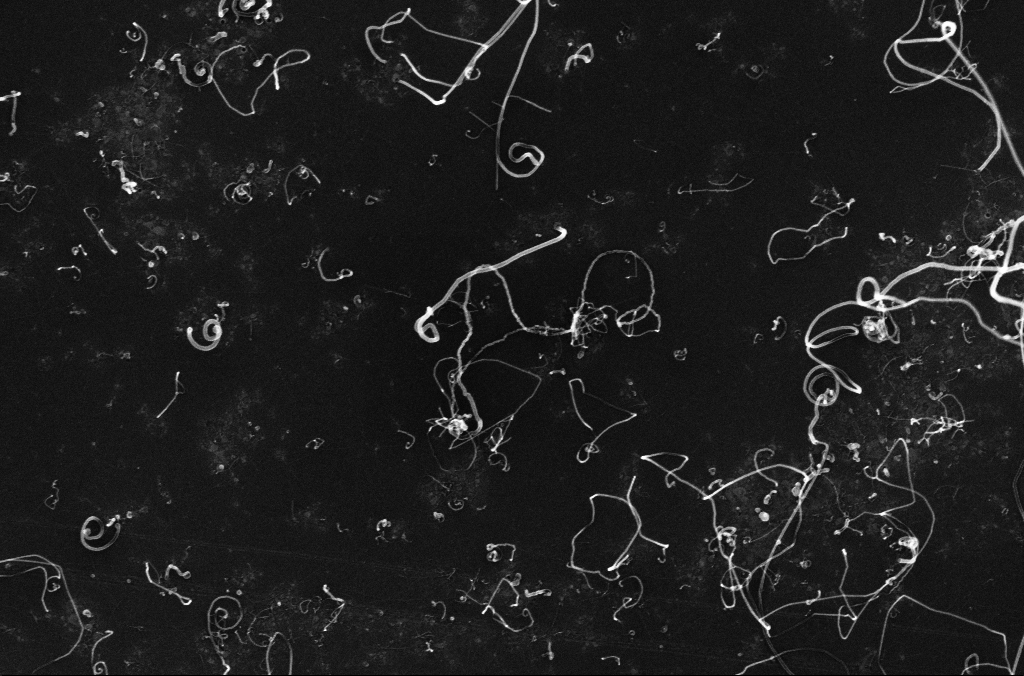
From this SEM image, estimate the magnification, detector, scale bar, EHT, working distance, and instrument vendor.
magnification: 60 K X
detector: InLens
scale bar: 1000 nm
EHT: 10 kV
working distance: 3.3 mm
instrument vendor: Zeiss